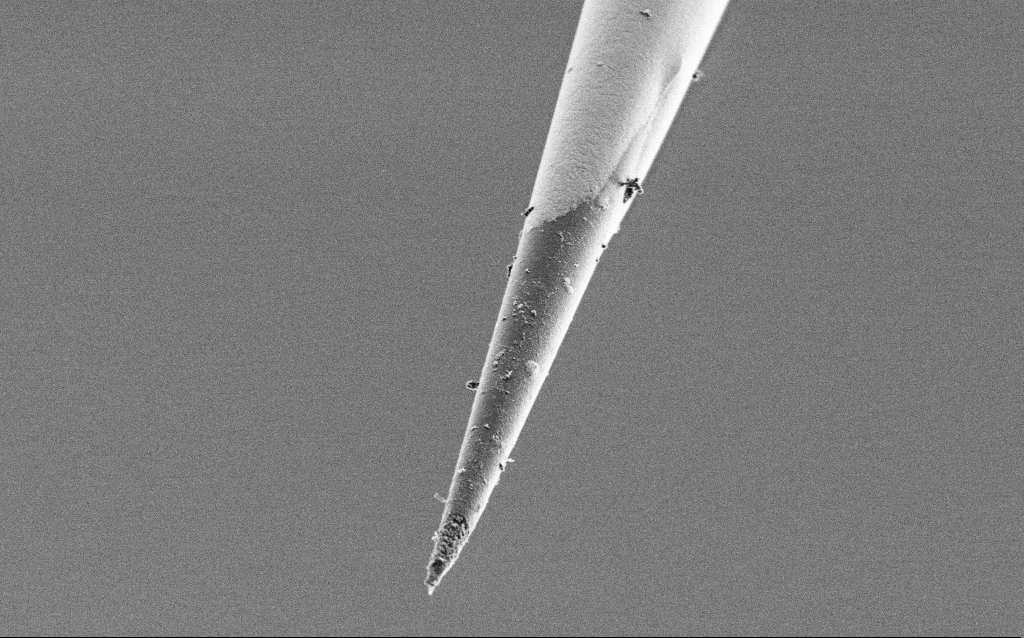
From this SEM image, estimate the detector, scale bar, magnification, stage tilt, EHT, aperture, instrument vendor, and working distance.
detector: SE2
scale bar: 1000 nm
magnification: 15 K X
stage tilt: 45°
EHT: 1 kV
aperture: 30 µm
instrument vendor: Zeiss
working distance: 6.5 mm